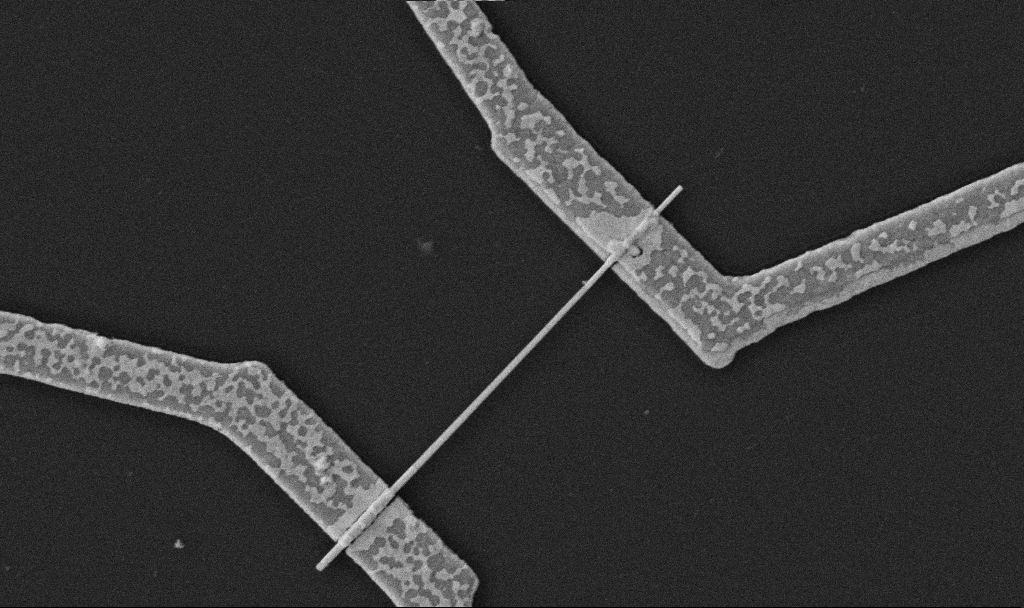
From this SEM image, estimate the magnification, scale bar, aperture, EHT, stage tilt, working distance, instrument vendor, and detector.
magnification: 30 K X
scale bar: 1000 nm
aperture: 30 µm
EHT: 5 kV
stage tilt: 0°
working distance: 10.7 mm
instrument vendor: Zeiss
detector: SE2